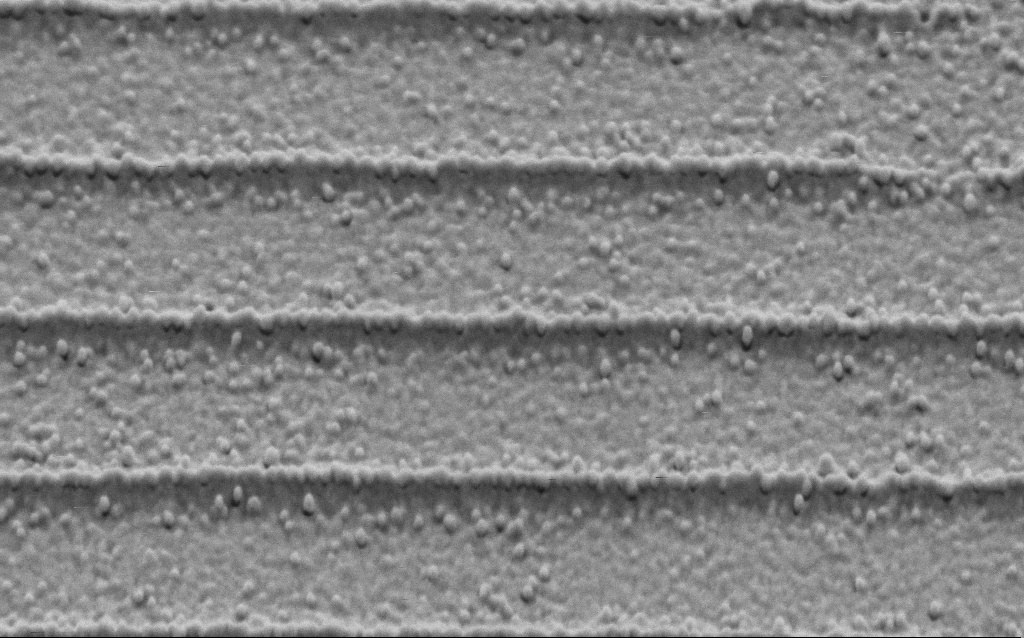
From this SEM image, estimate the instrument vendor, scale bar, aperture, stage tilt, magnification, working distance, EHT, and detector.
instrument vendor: Zeiss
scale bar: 200 nm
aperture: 30 µm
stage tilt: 45°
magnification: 106.83 K X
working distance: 4 mm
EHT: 3 kV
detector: SE2